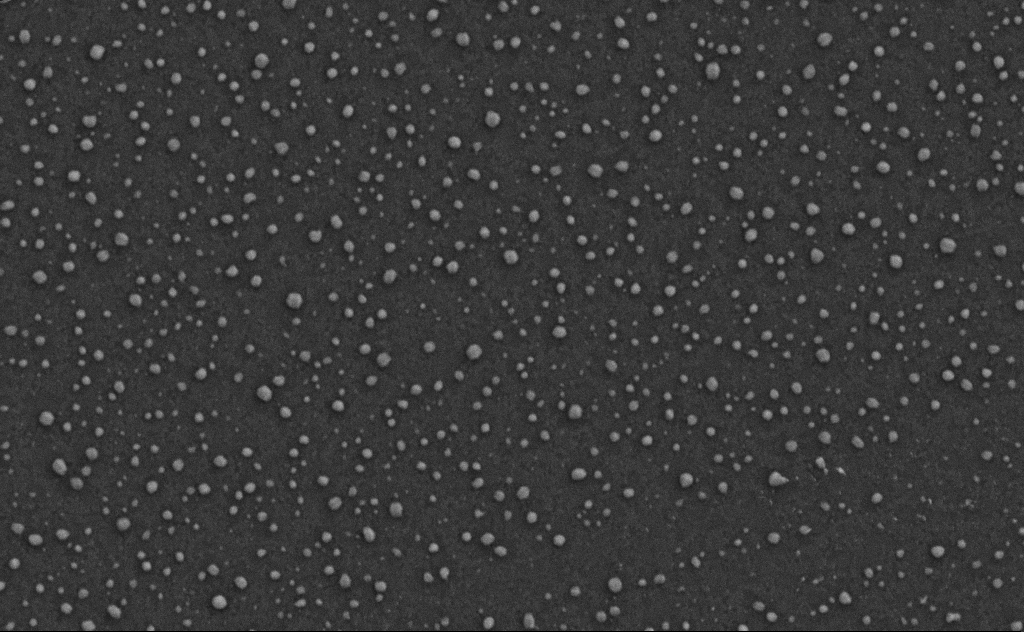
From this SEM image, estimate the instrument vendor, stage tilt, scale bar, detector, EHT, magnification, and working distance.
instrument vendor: Zeiss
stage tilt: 0°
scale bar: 1000 nm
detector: SE2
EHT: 5 kV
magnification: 40 K X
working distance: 6 mm